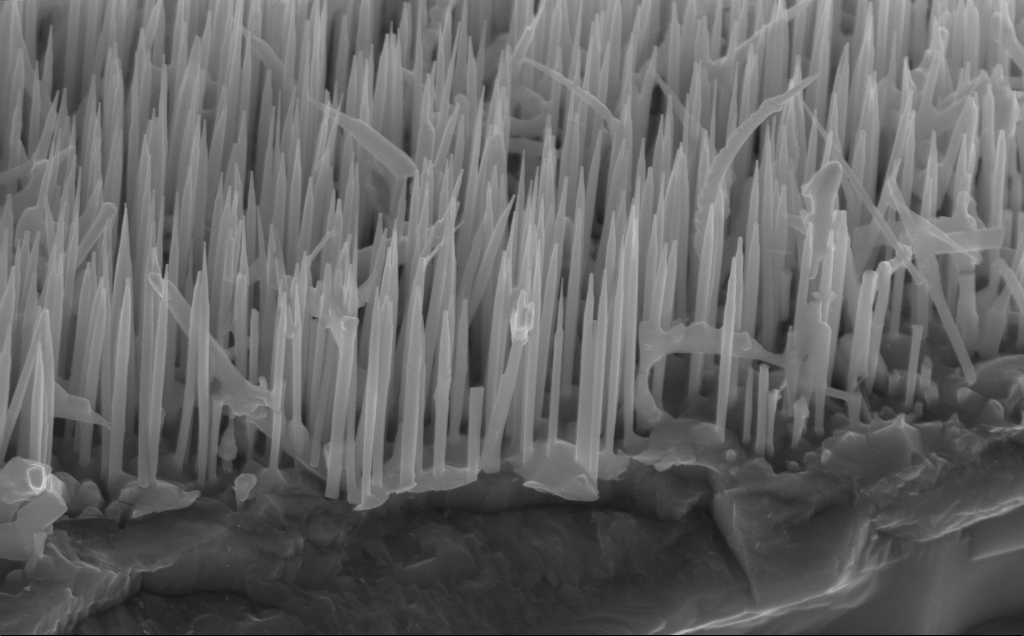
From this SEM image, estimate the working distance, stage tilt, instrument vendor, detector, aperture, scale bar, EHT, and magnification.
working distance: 5 mm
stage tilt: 45°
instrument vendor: Zeiss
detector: InLens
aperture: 30 µm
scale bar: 1000 nm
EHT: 12 kV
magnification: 40 K X